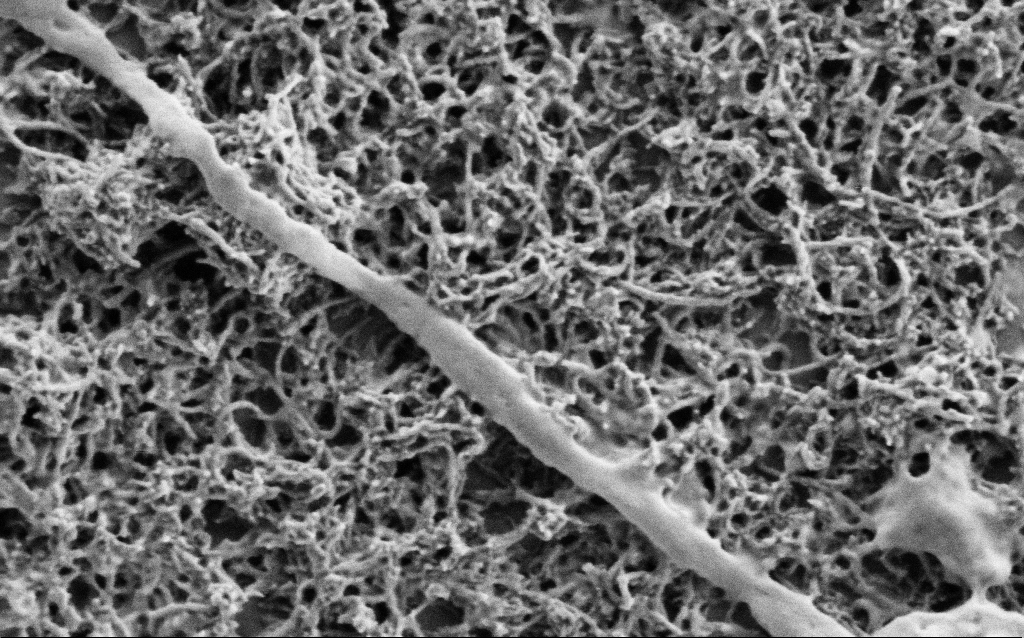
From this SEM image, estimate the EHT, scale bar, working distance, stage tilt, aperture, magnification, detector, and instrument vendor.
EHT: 1 kV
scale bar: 200 nm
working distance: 7 mm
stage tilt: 0°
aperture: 30 µm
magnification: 75 K X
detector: SE2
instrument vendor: Zeiss